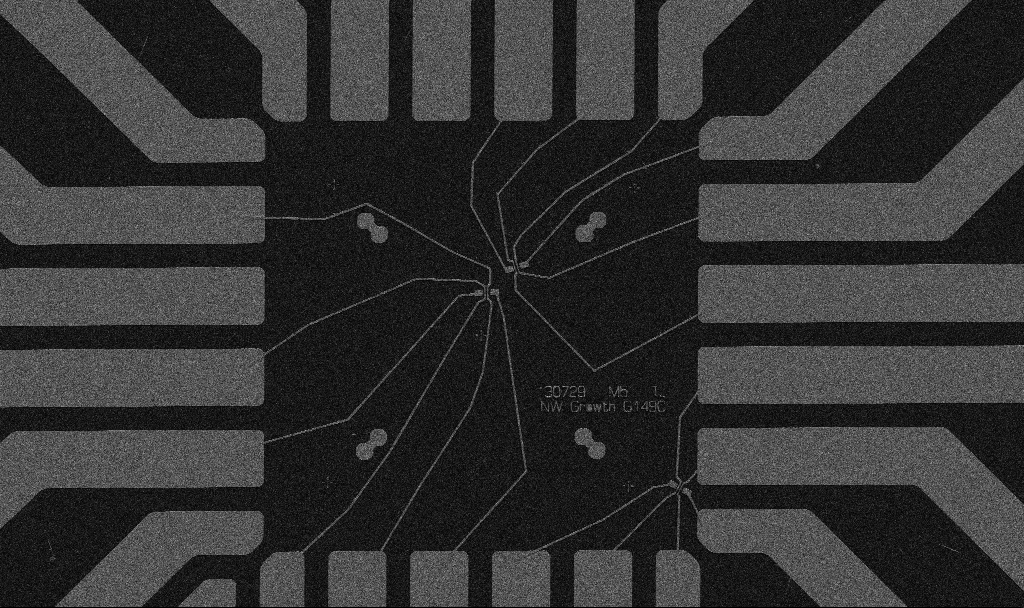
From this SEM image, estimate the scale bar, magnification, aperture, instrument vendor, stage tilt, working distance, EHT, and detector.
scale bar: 20000 nm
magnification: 1 K X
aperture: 30 µm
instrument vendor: Zeiss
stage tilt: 0°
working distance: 10.7 mm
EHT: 5 kV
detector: SE2